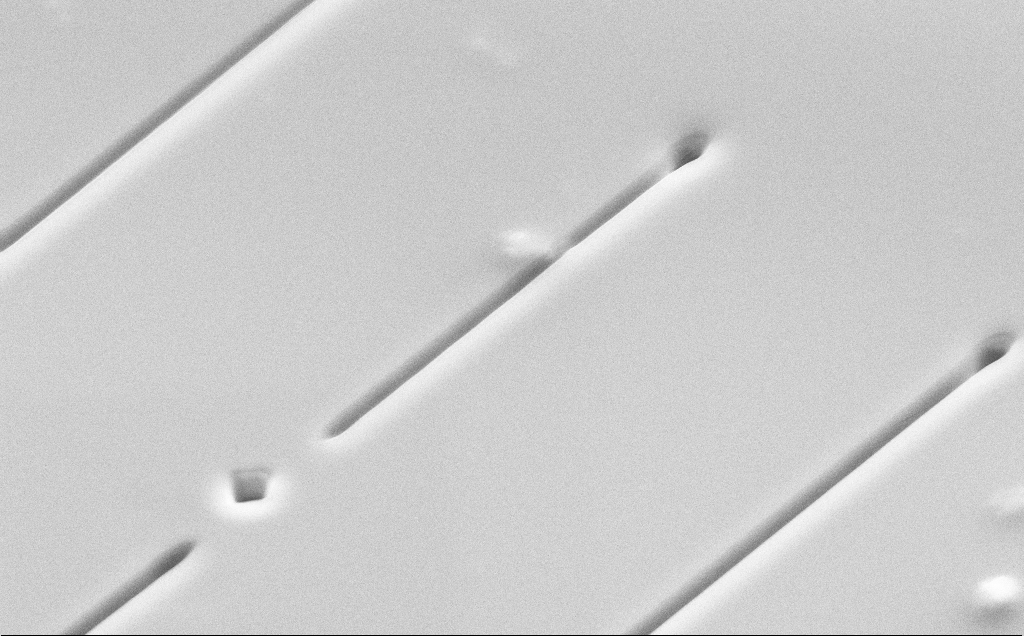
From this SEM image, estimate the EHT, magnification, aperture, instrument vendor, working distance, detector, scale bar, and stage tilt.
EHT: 10 kV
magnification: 7.7 K X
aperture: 30 µm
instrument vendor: Zeiss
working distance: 13 mm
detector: SE2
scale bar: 2000 nm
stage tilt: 45°